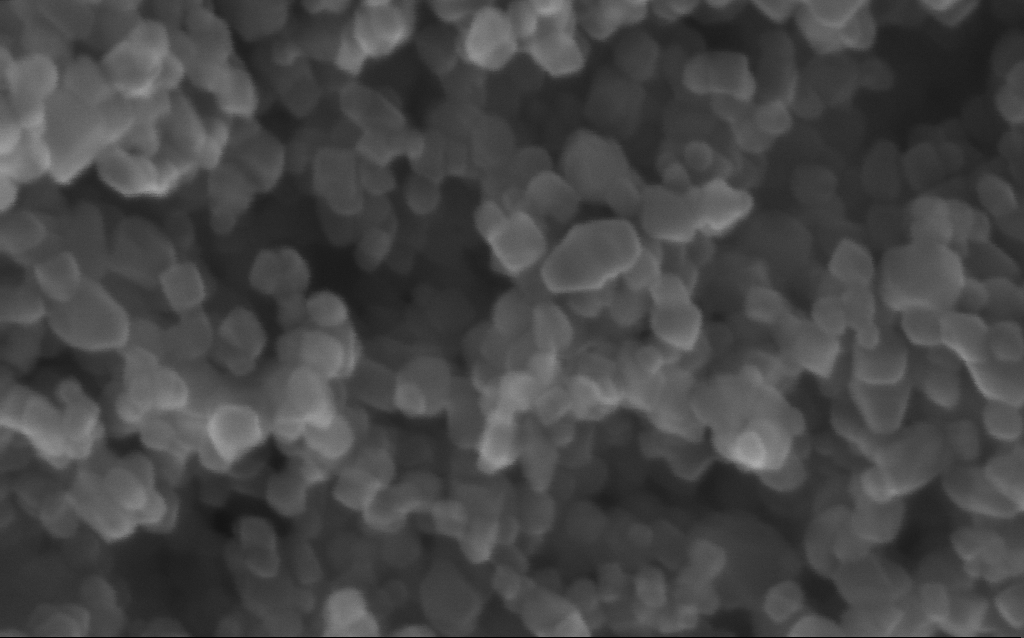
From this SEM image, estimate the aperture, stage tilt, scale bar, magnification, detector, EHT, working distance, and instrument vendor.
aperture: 30 µm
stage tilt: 0°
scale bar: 100 nm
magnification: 716 K X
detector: InLens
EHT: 10 kV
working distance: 2.7 mm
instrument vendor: Zeiss